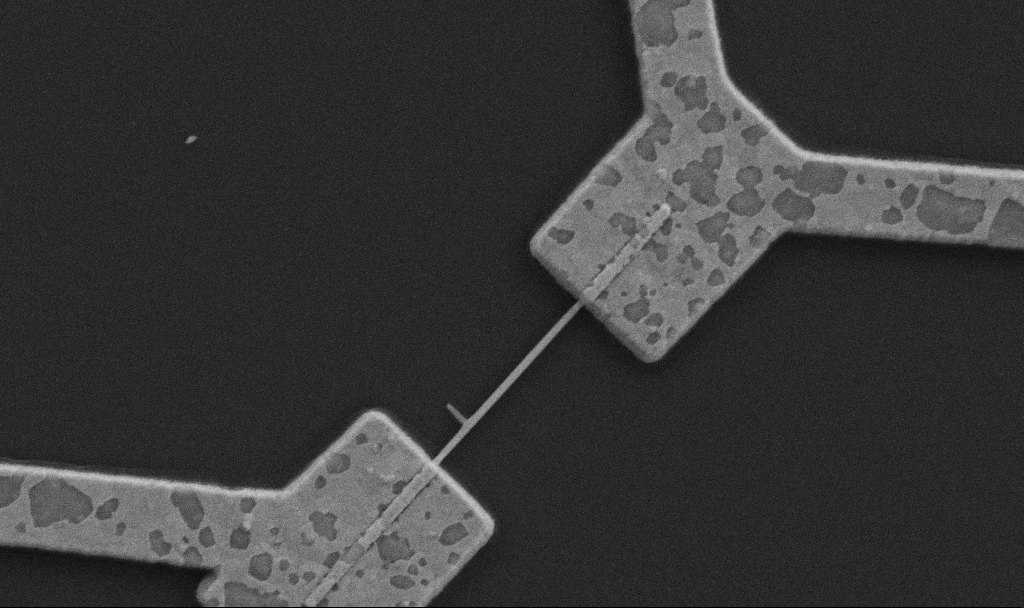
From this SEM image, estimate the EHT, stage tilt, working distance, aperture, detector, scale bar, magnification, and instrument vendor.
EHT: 5 kV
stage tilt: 0°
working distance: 10.7 mm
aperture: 30 µm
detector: SE2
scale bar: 1000 nm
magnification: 30 K X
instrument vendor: Zeiss